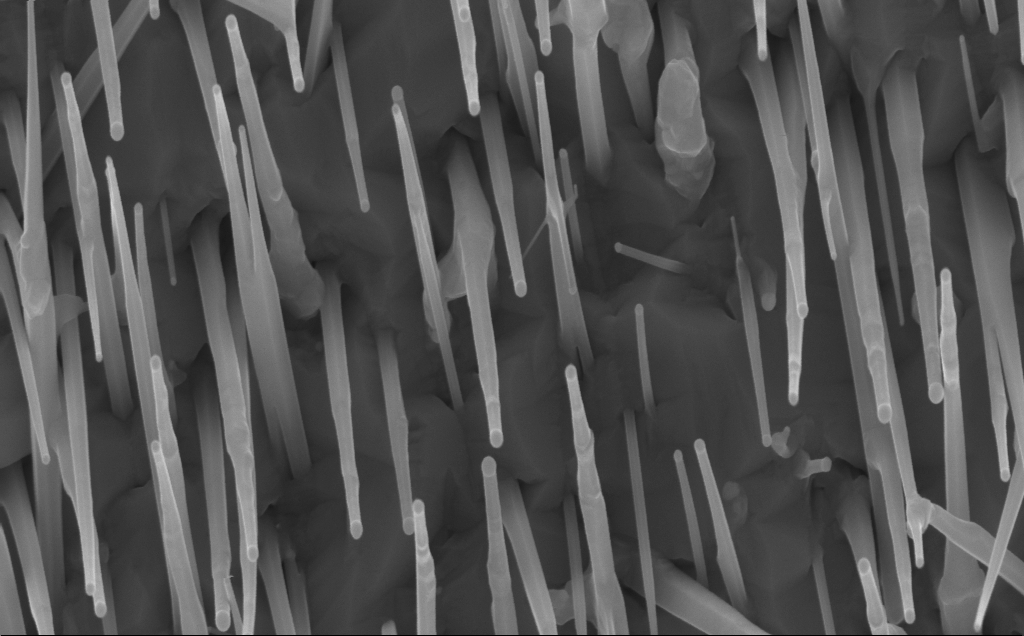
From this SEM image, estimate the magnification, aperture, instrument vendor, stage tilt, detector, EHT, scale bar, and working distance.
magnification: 80 K X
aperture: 30 µm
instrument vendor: Zeiss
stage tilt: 0°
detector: InLens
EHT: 10 kV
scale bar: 200 nm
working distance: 7 mm